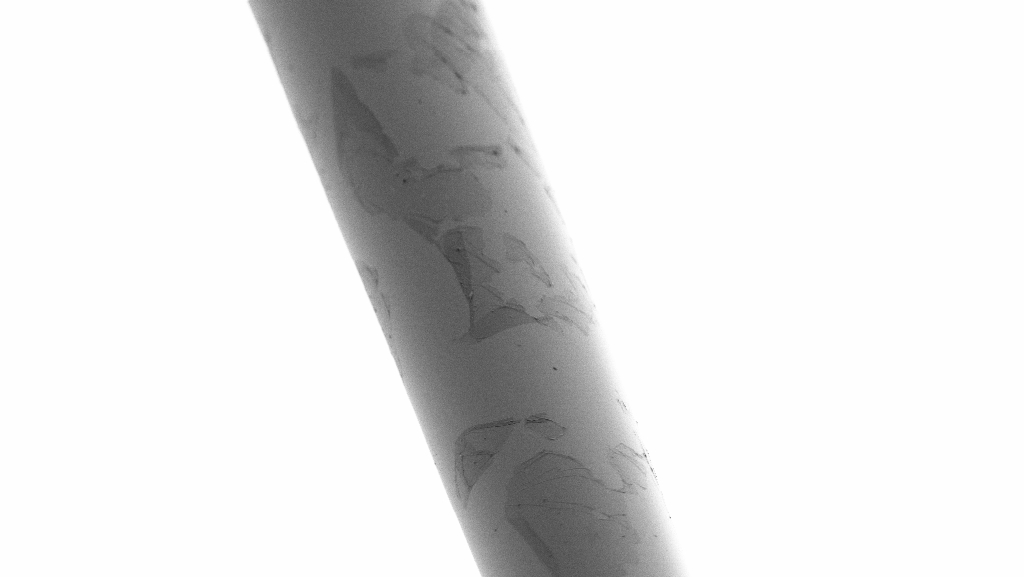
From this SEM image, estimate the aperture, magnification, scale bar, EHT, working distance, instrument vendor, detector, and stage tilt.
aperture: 30 µm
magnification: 2.5 K X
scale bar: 10000 nm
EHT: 1 kV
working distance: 4 mm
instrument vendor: Zeiss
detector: SE2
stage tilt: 45°